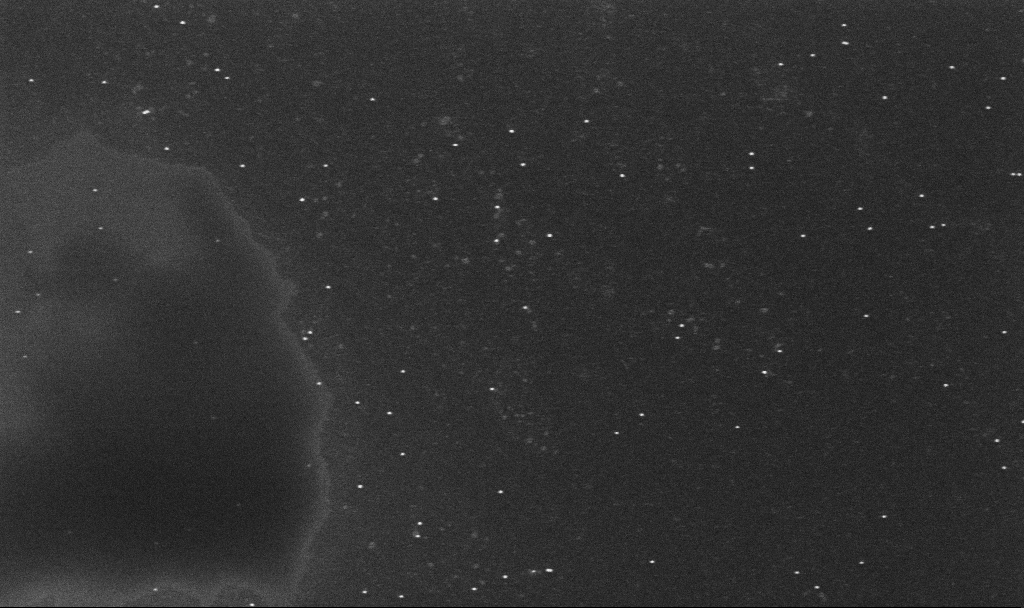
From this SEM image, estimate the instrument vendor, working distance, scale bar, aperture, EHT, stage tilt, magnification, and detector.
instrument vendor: Zeiss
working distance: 3.2 mm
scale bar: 200 nm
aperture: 30 µm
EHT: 10 kV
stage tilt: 0°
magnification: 100 K X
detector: InLens